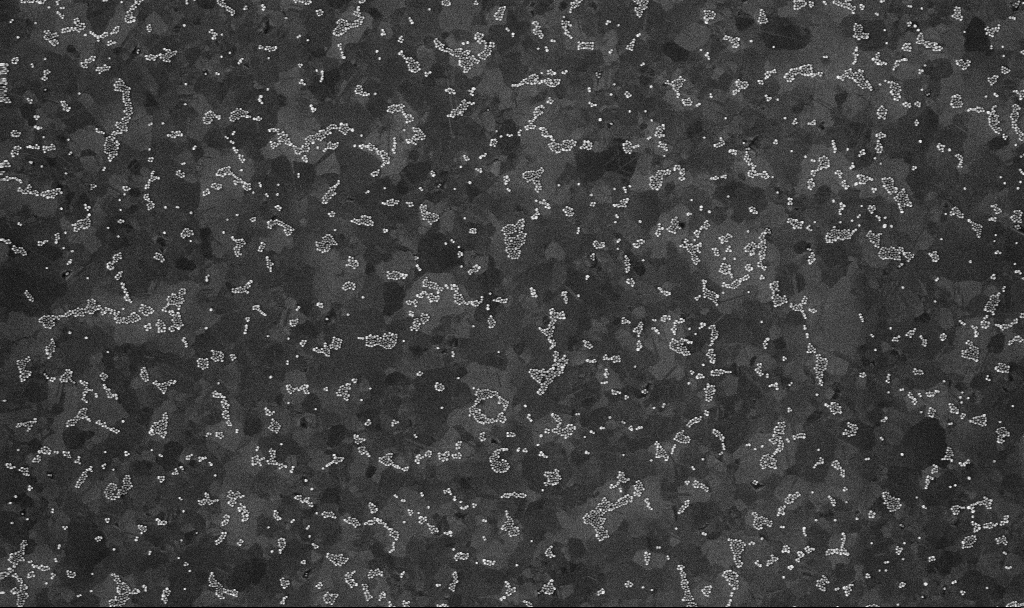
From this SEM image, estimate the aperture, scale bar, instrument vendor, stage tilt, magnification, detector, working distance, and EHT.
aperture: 30 µm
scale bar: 1000 nm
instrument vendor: Zeiss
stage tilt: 0°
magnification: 50 K X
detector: InLens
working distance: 3.3 mm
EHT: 10 kV